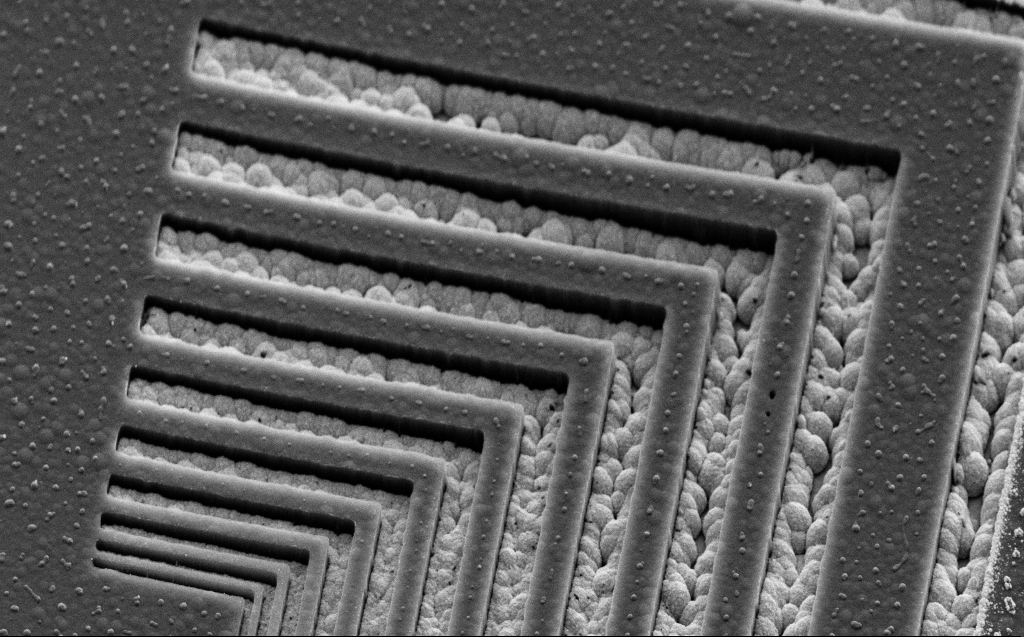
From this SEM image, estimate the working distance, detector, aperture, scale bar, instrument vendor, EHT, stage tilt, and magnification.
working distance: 9 mm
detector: SE2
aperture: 30 µm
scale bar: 2000 nm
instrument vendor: Zeiss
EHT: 5 kV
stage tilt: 45°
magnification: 20.38 K X